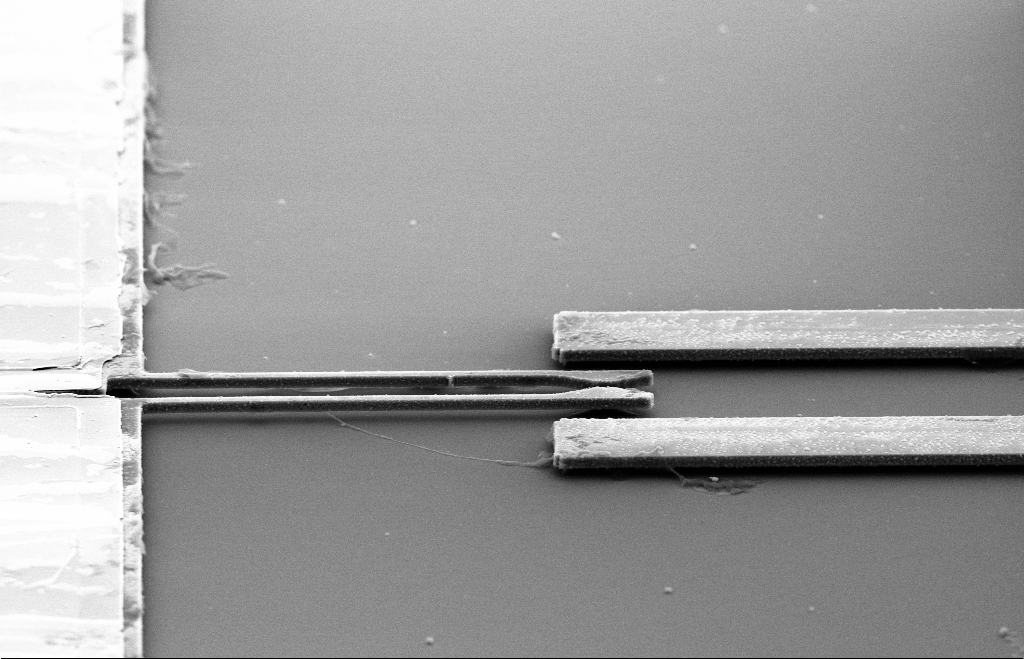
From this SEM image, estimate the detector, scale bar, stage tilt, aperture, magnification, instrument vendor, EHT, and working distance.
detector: SE2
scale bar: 10000 nm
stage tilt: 66.4°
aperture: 30 µm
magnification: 1.88 K X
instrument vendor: Zeiss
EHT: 10 kV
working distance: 12 mm